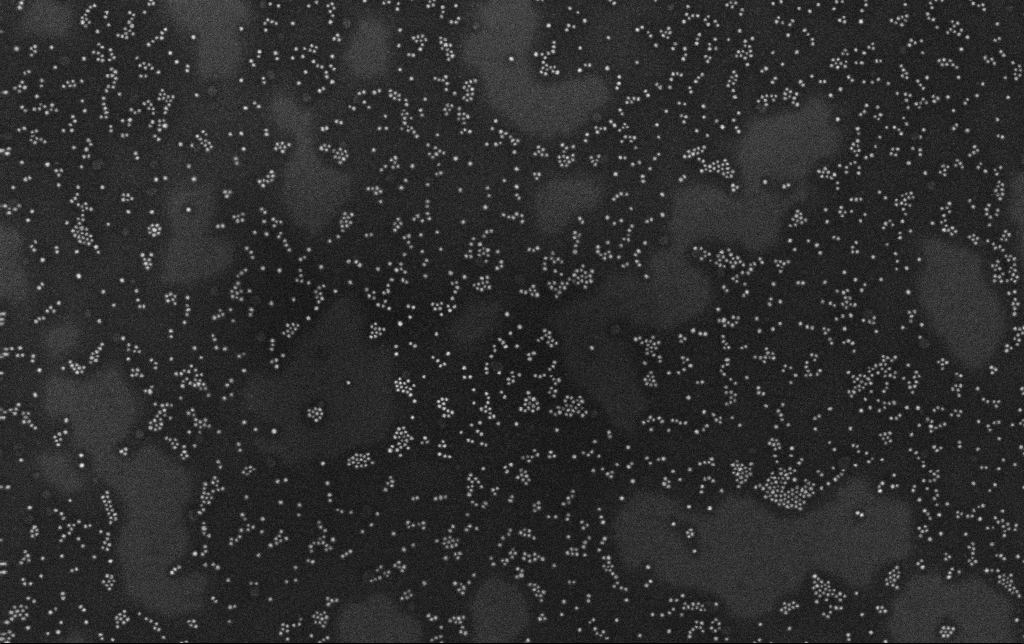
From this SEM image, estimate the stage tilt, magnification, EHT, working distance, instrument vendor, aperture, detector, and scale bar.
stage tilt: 0°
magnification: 100 K X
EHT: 10 kV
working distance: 3.4 mm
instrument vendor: Zeiss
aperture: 30 µm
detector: InLens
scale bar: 200 nm